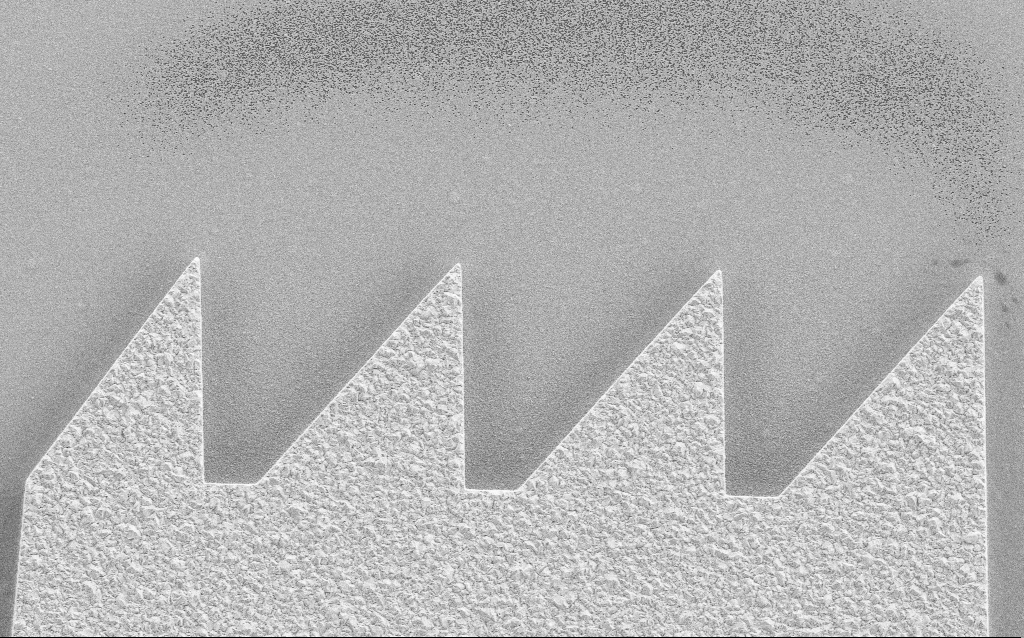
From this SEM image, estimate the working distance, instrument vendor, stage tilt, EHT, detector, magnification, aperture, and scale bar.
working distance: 4.5 mm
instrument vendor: Zeiss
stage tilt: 0°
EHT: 3 kV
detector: SE2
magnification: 8.46 K X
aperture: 30 µm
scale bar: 2000 nm